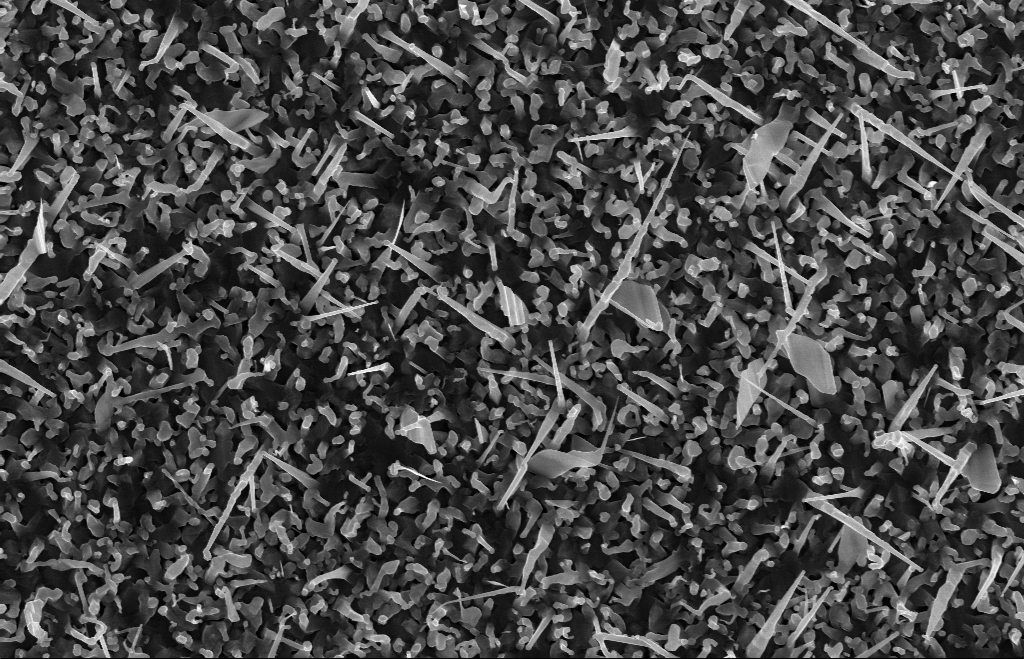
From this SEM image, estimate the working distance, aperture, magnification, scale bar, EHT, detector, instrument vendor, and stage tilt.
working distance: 8 mm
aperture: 30 µm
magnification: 20 K X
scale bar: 1000 nm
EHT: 10 kV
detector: InLens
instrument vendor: Zeiss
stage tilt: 0°